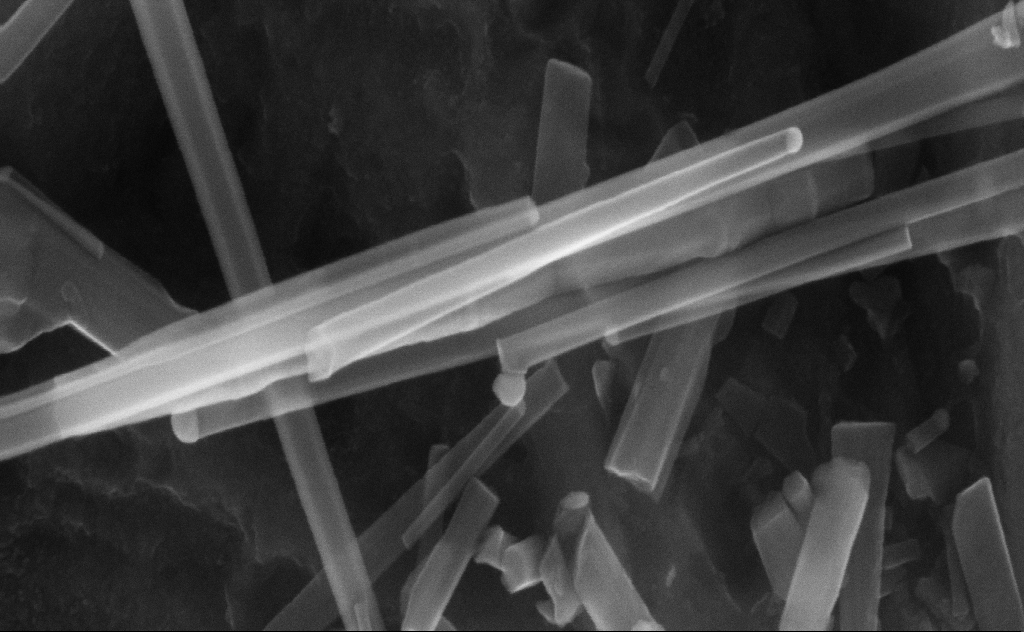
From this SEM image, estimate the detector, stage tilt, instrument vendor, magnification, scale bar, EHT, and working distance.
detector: InLens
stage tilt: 0°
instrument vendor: Zeiss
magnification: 200.79 K X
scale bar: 200 nm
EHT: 20 kV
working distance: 8 mm